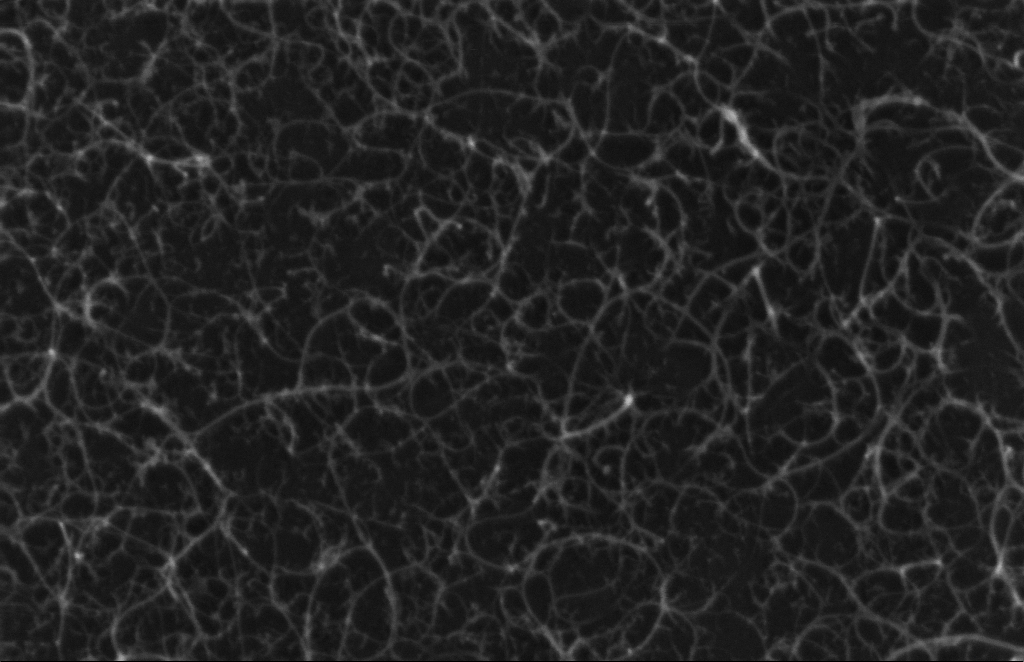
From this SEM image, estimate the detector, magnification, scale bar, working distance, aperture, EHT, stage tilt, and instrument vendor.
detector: InLens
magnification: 239.21 K X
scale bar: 100 nm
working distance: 5 mm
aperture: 20 µm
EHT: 5 kV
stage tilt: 0°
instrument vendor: Zeiss